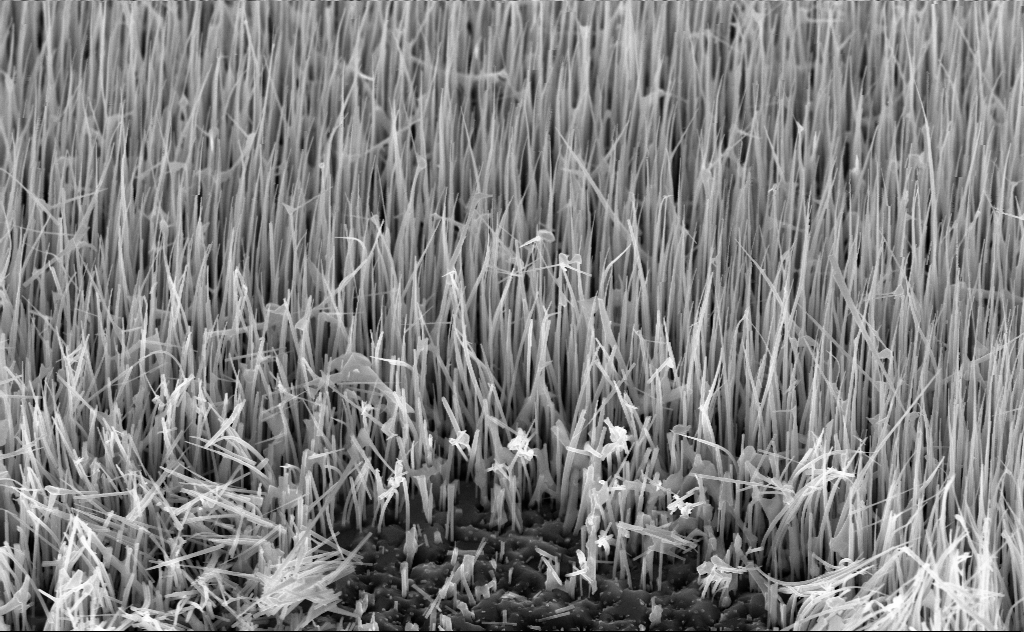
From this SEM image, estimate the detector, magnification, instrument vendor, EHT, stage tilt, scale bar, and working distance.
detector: InLens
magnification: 28.81 K X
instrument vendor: Zeiss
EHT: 10 kV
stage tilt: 45°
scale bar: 2000 nm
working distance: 6 mm